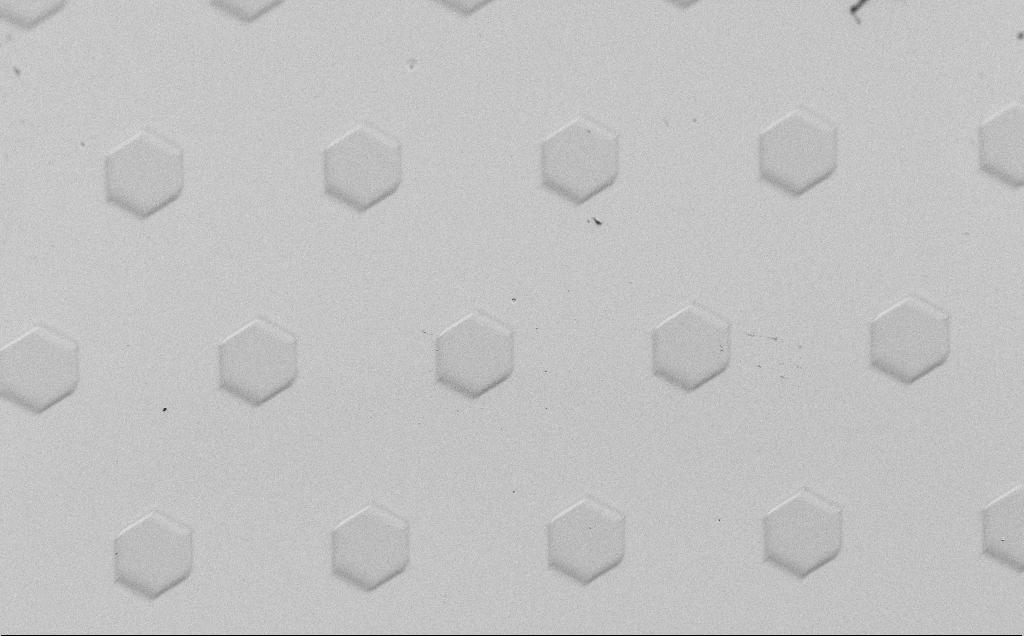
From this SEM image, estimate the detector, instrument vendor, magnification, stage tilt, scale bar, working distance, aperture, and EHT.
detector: SE2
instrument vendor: Zeiss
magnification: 0.534 K X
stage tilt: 45°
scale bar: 100000 nm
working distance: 6 mm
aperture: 30 µm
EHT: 1.5 kV